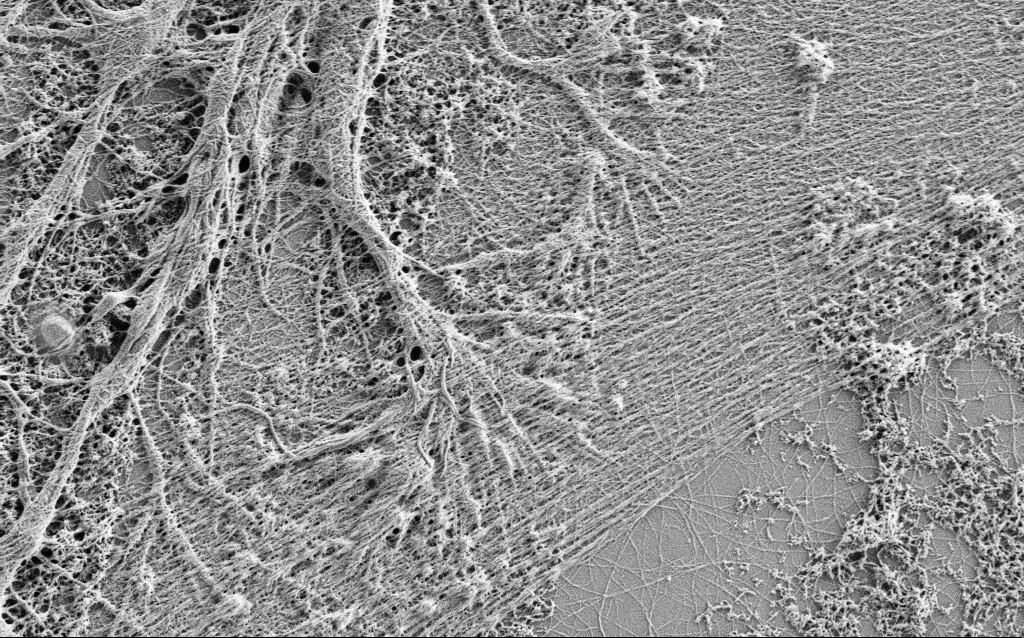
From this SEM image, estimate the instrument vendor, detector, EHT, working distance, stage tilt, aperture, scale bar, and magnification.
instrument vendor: Zeiss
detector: SE2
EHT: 1 kV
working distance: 4 mm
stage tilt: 0°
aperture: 30 µm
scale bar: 2000 nm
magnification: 10 K X